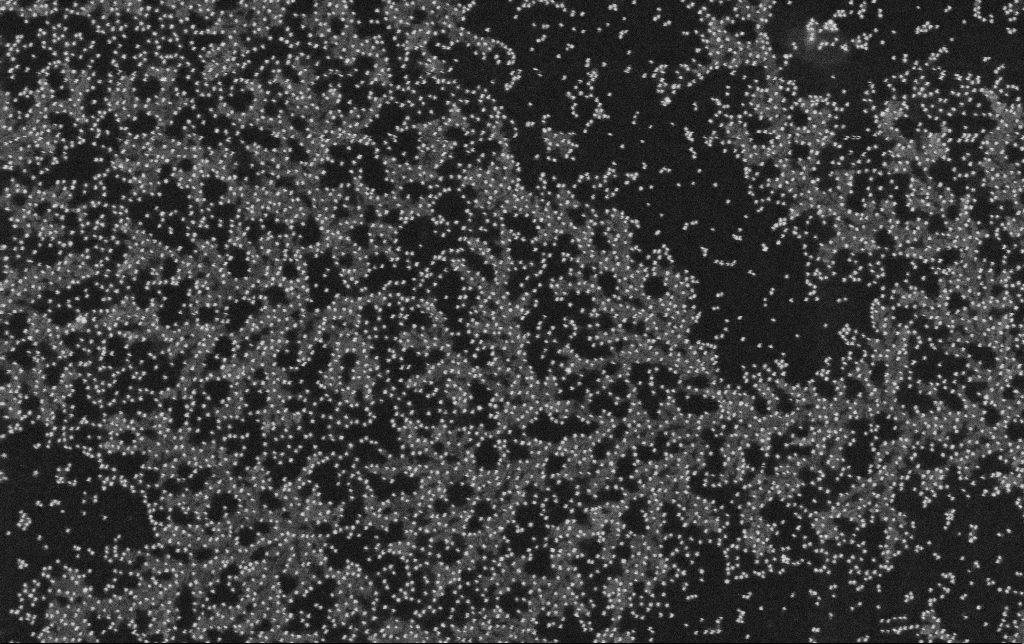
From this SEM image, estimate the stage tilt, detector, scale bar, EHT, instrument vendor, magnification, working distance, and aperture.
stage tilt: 0°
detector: SE2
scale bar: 200 nm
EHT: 10 kV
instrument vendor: Zeiss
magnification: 100 K X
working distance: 11.3 mm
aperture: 30 µm